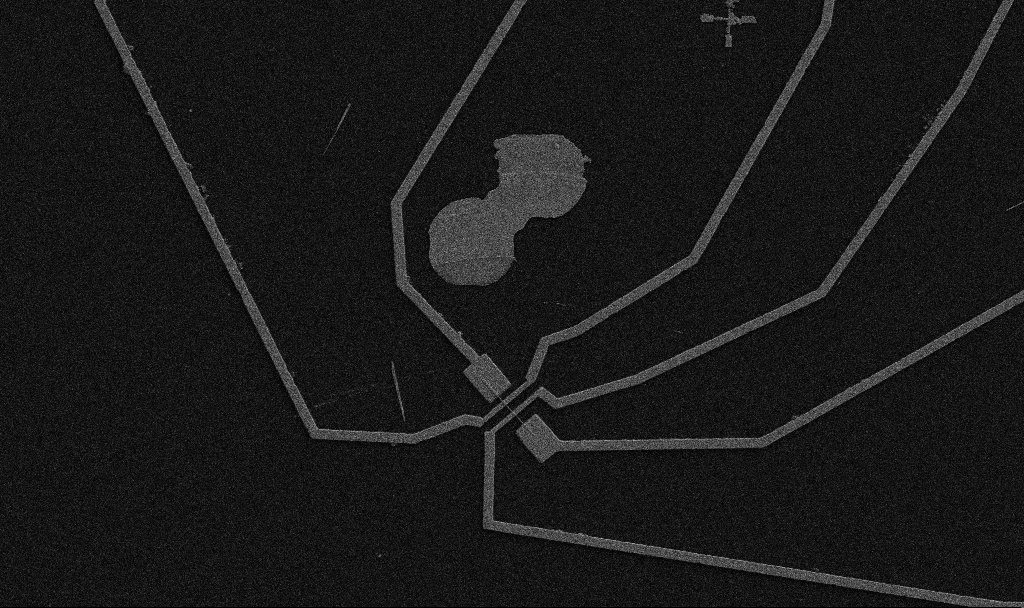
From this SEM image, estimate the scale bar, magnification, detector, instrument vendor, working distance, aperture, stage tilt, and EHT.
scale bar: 10000 nm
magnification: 5 K X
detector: SE2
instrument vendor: Zeiss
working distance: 10.7 mm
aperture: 30 µm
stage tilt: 0°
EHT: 5 kV